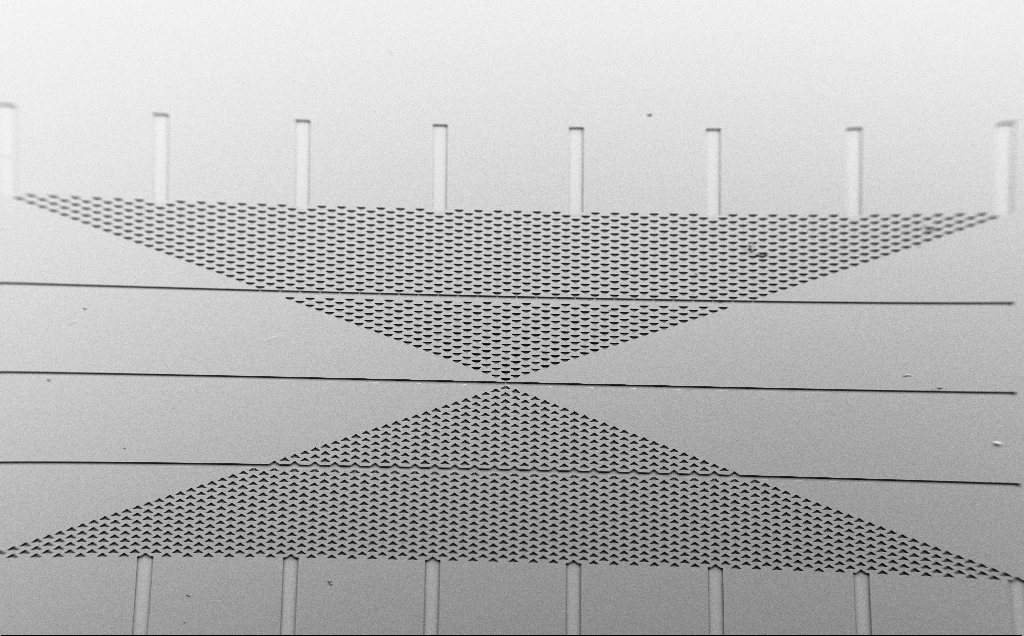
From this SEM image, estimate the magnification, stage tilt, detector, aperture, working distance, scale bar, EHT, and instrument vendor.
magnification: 0.096 K X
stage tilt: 40°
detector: SE2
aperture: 30 µm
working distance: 8 mm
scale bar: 200000 nm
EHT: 5 kV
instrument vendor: Zeiss